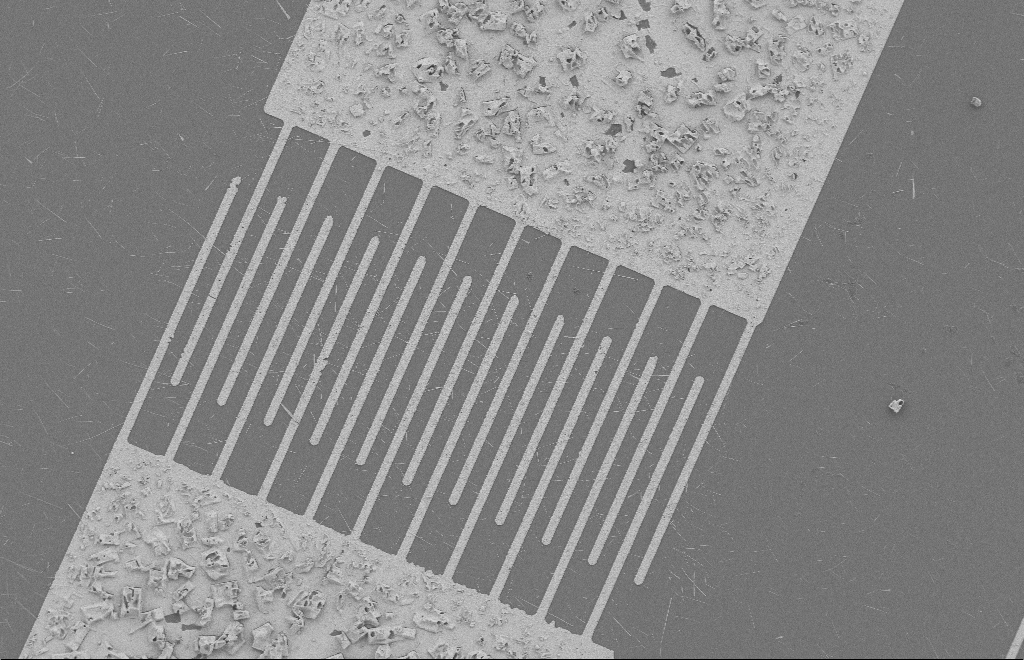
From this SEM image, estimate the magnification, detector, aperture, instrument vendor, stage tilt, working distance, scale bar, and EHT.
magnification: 1.32 K X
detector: SE2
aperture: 20 µm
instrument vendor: Zeiss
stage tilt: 0°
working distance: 8 mm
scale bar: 20000 nm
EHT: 2 kV